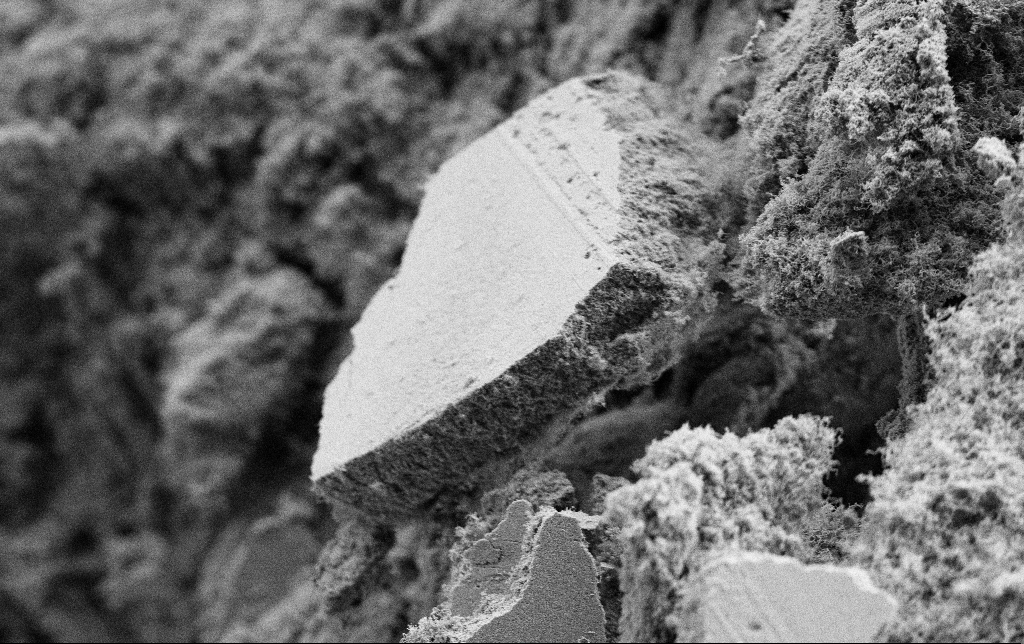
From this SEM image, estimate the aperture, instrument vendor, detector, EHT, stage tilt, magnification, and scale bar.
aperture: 30 µm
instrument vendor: Zeiss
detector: SE2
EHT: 2 kV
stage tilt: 0°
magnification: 5 K X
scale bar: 10000 nm